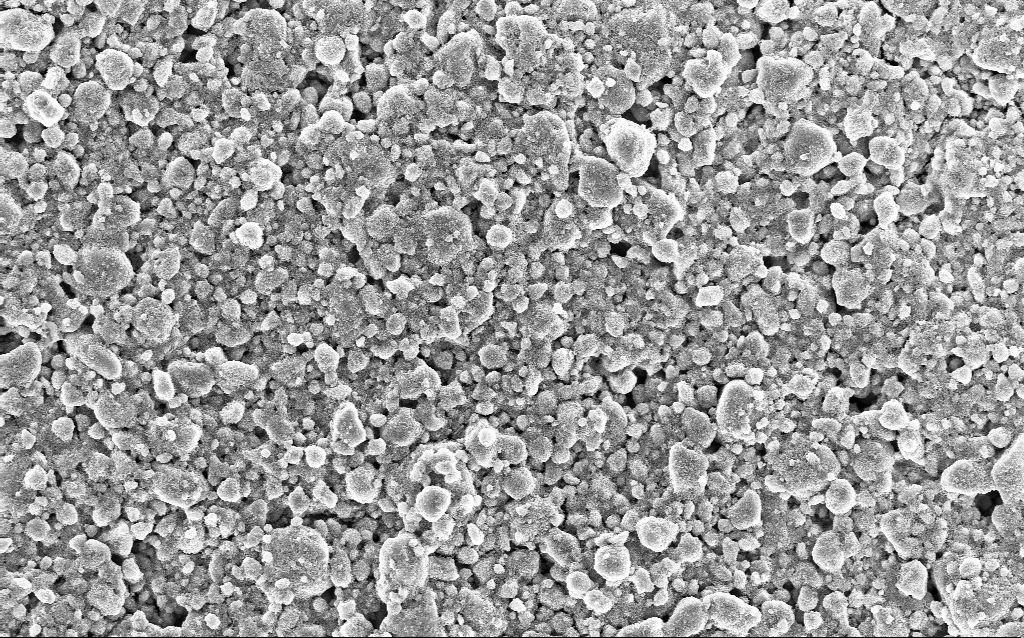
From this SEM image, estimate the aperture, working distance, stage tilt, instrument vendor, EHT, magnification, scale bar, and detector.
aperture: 30 µm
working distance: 3.8 mm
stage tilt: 0°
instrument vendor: Zeiss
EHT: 10 kV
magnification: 3.12 K X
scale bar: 10000 nm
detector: InLens